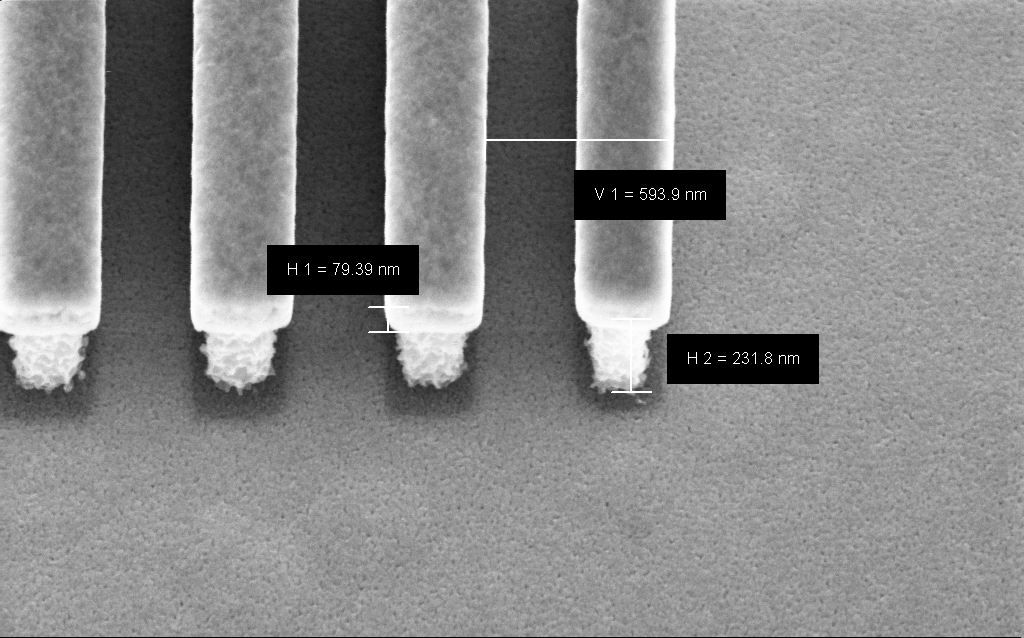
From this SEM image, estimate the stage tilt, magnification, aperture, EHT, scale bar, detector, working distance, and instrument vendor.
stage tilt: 30°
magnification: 115.62 K X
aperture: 30 µm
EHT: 5 kV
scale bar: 200 nm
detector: InLens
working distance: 4.1 mm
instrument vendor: Zeiss